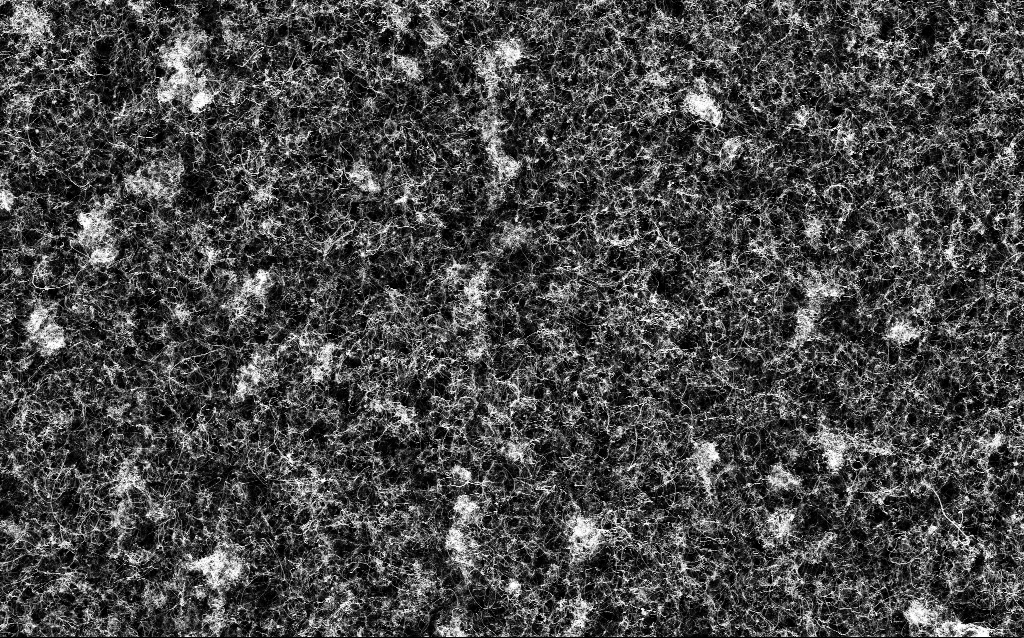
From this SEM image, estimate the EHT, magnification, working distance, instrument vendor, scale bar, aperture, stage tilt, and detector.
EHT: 5 kV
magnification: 10 K X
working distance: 3.3 mm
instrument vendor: Zeiss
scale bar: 2000 nm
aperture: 30 µm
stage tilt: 0°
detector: InLens